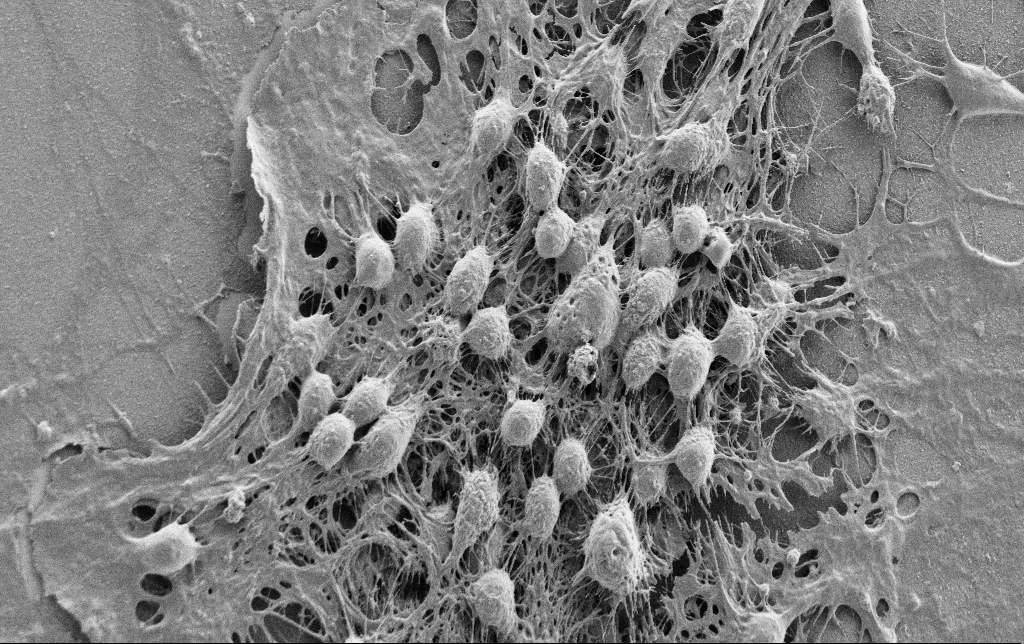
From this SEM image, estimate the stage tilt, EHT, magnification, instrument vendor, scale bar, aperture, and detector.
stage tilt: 0°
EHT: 0.9 kV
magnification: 3 K X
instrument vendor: Zeiss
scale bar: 10000 nm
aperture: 30 µm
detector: SE2